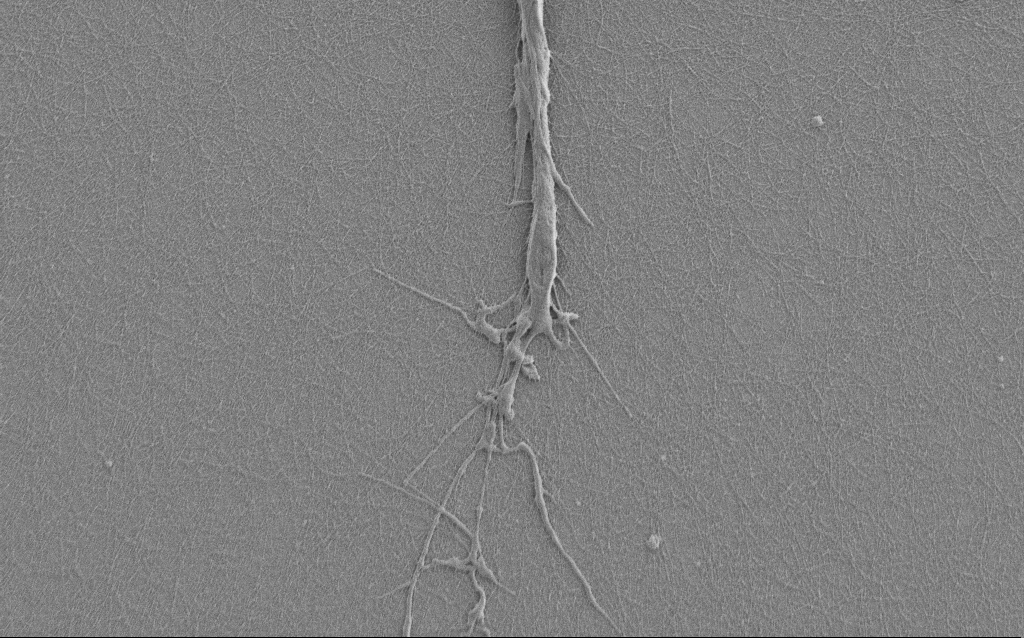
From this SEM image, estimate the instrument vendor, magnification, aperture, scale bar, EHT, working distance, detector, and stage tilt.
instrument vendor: Zeiss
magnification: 7.5 K X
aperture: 30 µm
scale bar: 2000 nm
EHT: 1 kV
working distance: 6 mm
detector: SE2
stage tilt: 0°